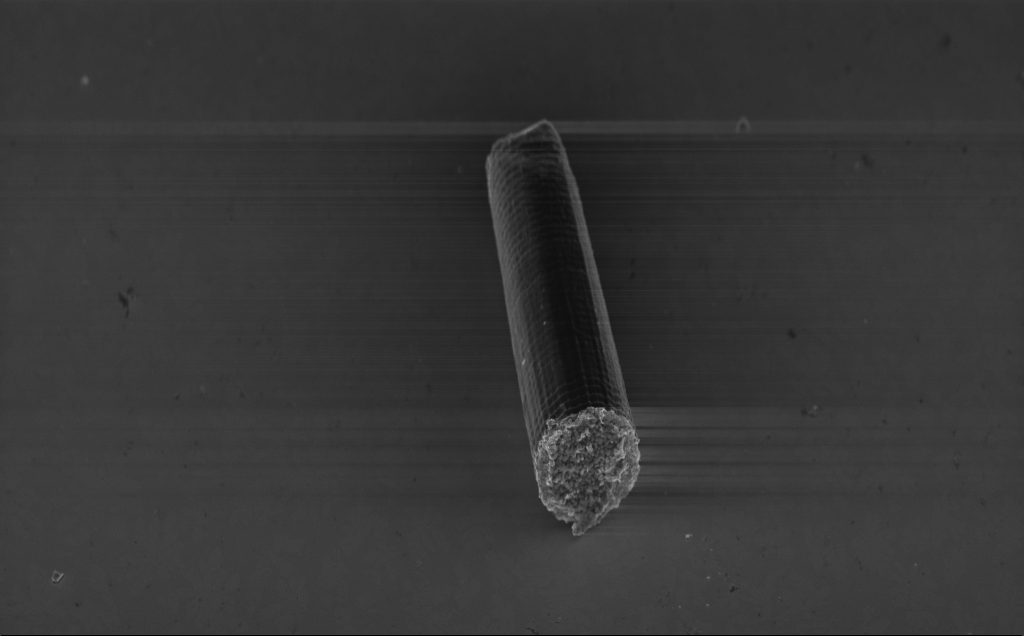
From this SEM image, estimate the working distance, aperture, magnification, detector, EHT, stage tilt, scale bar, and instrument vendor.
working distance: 7 mm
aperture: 30 µm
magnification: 4.33 K X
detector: InLens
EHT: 5 kV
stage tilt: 45°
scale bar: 10000 nm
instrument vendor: Zeiss